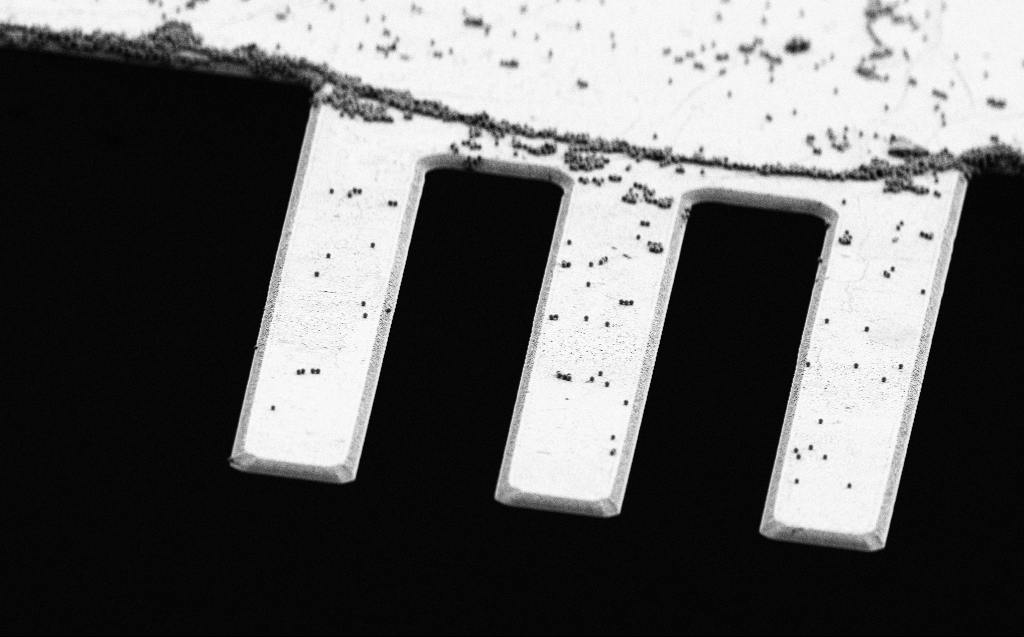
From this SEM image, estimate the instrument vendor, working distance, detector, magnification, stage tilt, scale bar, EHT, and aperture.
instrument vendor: Zeiss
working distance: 7 mm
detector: SE2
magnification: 2.47 K X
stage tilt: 64.8°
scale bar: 20000 nm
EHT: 3 kV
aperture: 30 µm